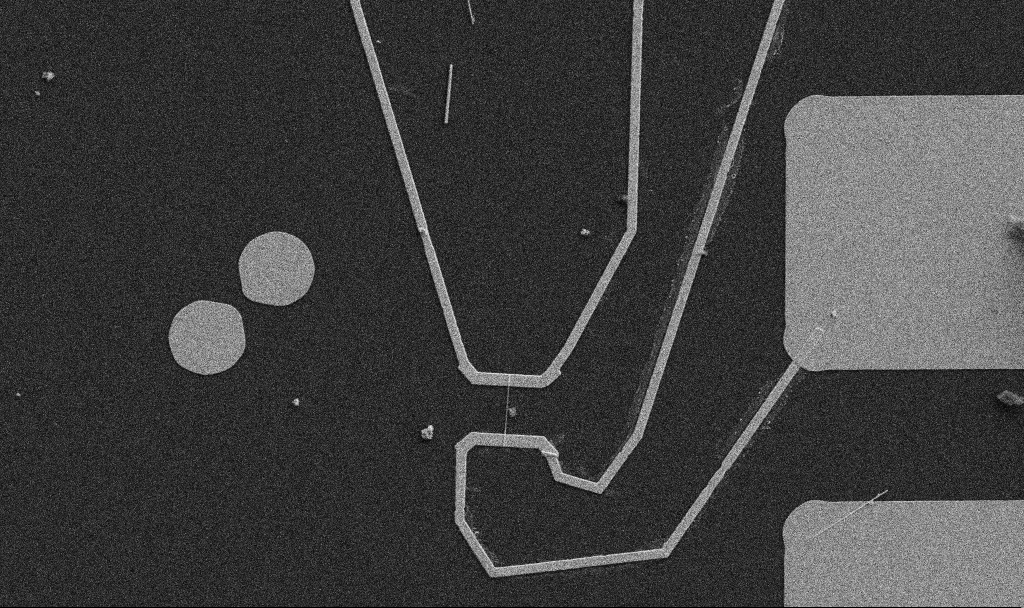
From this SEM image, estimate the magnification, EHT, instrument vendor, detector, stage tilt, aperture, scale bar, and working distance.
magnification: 5 K X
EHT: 5 kV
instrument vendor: Zeiss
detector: SE2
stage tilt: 0°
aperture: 30 µm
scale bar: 10000 nm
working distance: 10.7 mm